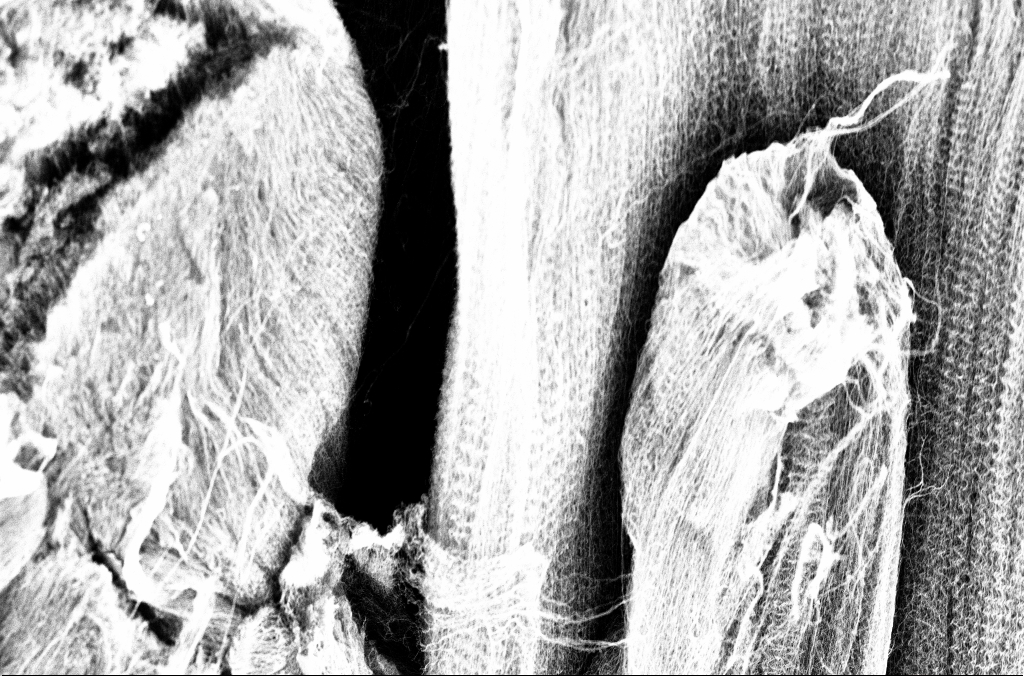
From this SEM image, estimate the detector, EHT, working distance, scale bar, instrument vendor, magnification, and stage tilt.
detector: InLens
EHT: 3 kV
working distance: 3.4 mm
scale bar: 10000 nm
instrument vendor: Zeiss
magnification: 5 K X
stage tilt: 45°